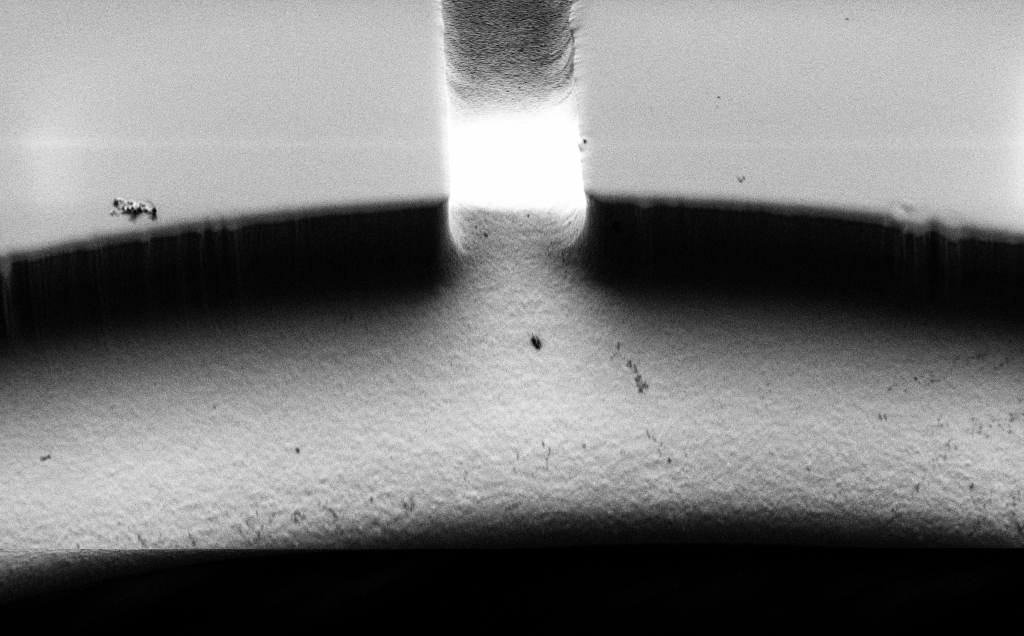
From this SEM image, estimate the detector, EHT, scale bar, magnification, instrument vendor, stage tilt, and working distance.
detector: SE2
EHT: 0.8 kV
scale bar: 20000 nm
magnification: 1.06 K X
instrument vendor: Zeiss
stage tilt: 45°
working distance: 7 mm